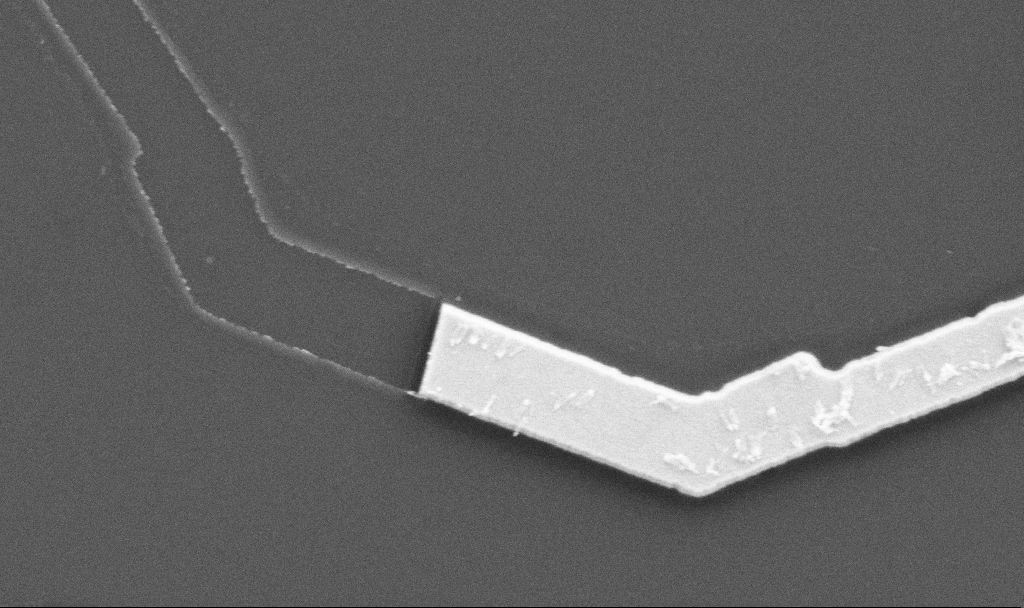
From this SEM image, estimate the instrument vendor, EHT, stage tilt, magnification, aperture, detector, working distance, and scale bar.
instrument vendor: Zeiss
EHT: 5 kV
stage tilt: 0°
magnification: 36.18 K X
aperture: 30 µm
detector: SE2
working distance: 10.7 mm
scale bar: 2000 nm